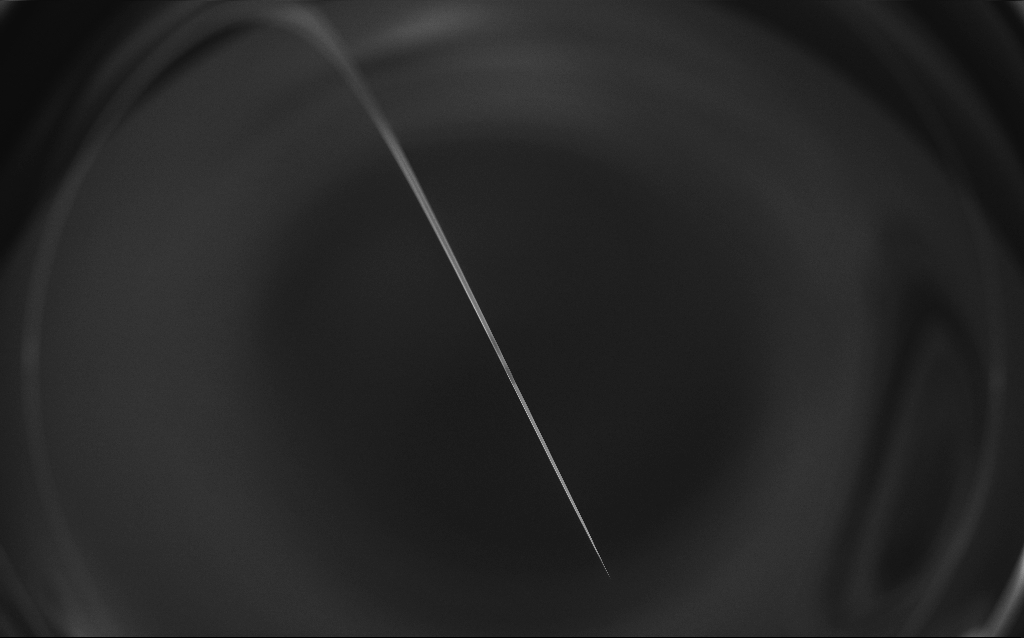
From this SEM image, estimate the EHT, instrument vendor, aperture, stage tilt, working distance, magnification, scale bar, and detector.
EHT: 1 kV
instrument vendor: Zeiss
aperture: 30 µm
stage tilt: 45°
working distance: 7 mm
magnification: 0.1 K X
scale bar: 200000 nm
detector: InLens